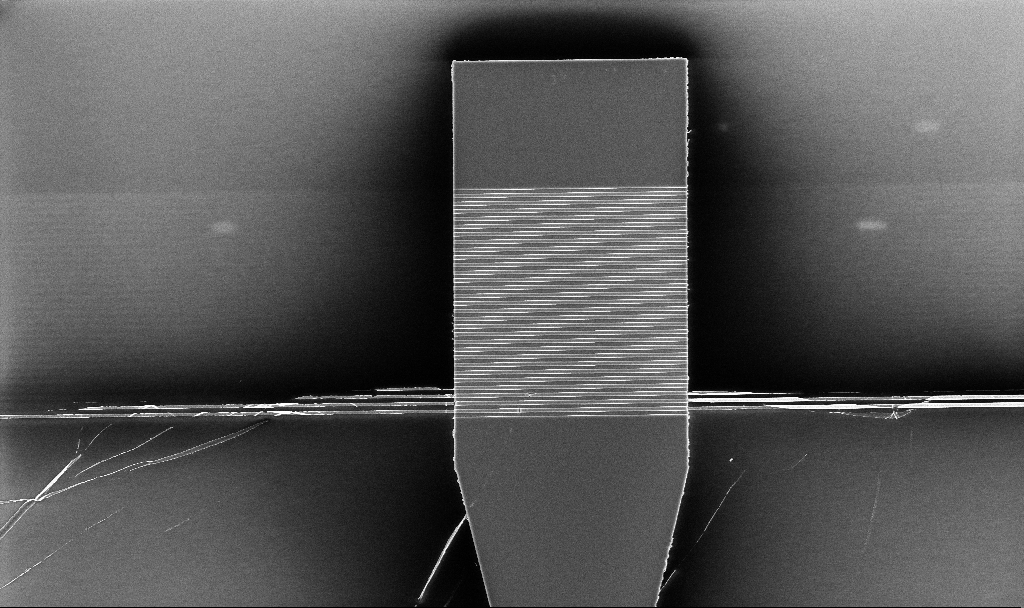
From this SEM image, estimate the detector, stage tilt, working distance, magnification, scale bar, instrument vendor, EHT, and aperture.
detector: InLens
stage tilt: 0°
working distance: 5.2 mm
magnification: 4.34 K X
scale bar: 10000 nm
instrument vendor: Zeiss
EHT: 5 kV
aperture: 30 µm